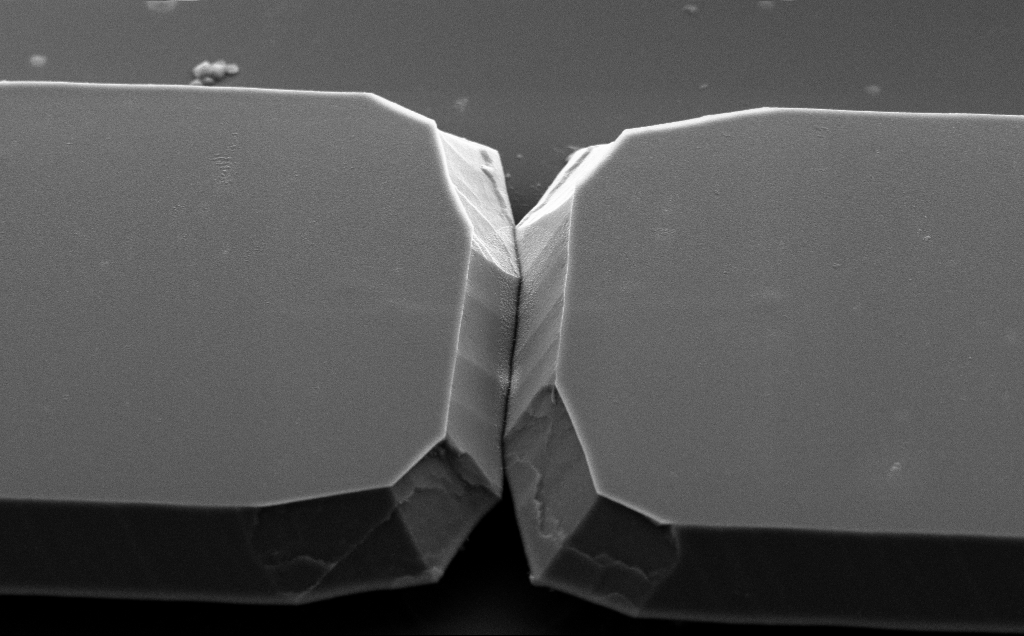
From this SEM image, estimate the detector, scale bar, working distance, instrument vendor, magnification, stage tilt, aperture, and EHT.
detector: SE2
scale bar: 1000 nm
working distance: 10 mm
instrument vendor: Zeiss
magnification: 12.63 K X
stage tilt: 50°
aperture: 30 µm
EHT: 5 kV